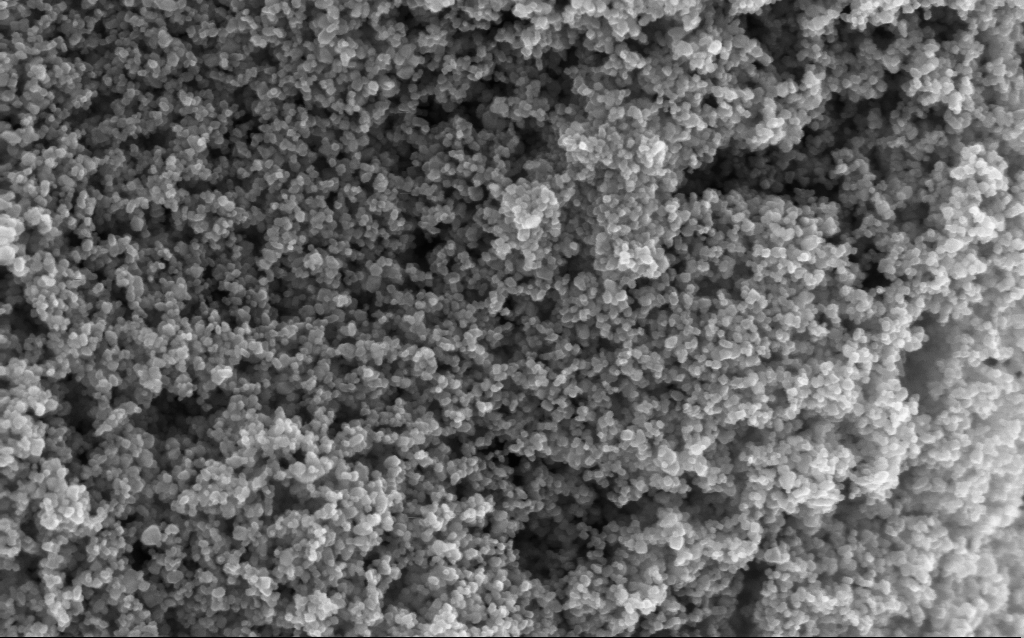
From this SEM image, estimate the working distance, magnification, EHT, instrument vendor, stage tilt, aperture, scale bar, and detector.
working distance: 3 mm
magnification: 130 K X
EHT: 5 kV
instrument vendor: Zeiss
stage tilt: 0°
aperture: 30 µm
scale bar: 100 nm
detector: InLens